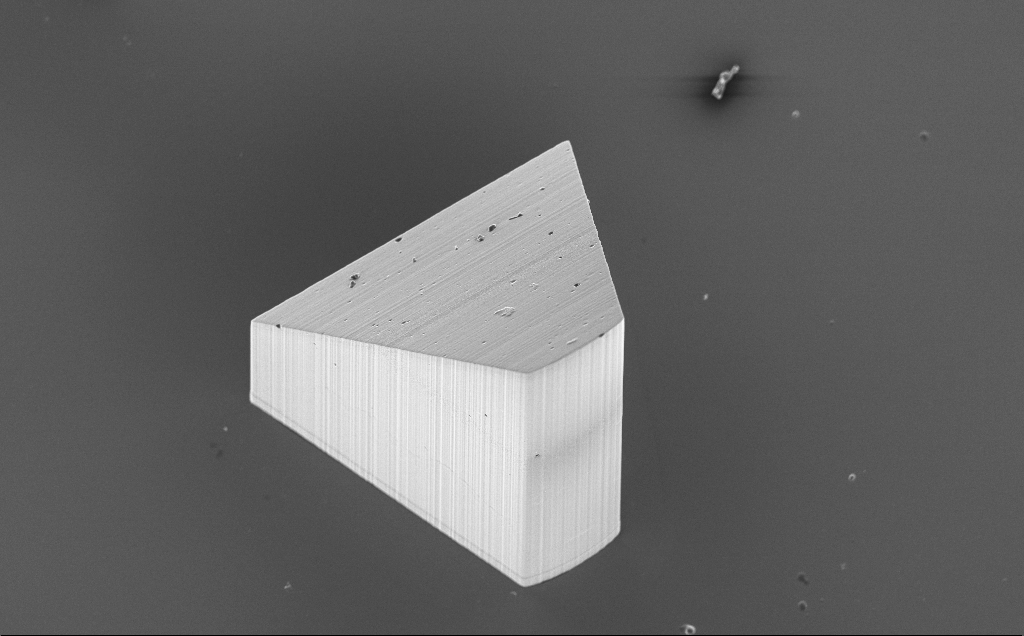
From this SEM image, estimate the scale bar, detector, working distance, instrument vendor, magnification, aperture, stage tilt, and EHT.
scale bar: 100000 nm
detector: InLens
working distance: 9 mm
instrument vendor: Zeiss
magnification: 0.347 K X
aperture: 30 µm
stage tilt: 20°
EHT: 10 kV